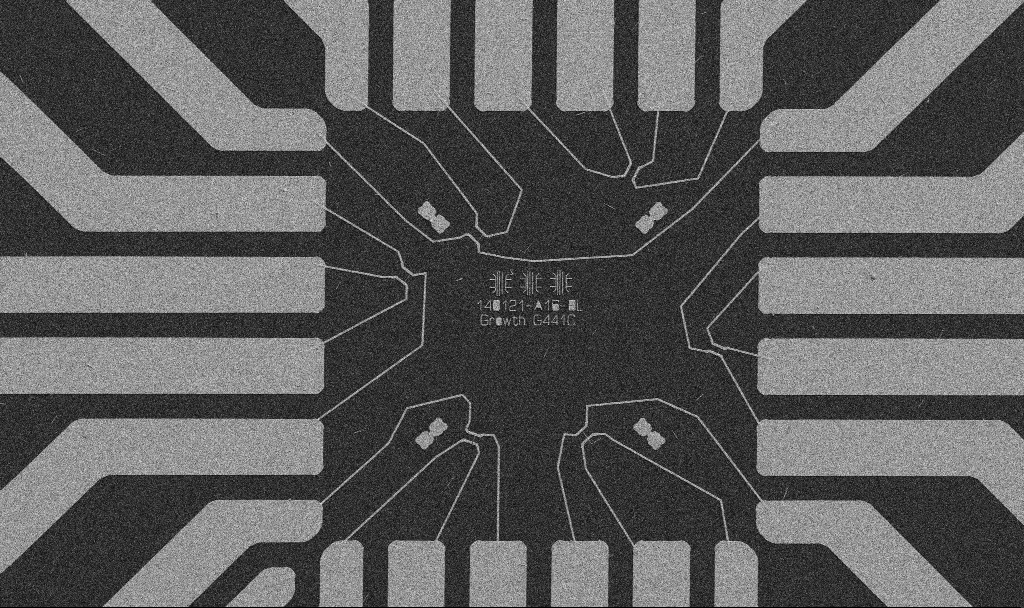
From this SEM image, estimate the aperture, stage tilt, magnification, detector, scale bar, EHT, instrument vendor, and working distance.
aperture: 30 µm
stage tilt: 0°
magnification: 1 K X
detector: SE2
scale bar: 20000 nm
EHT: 5 kV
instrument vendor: Zeiss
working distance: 10.7 mm